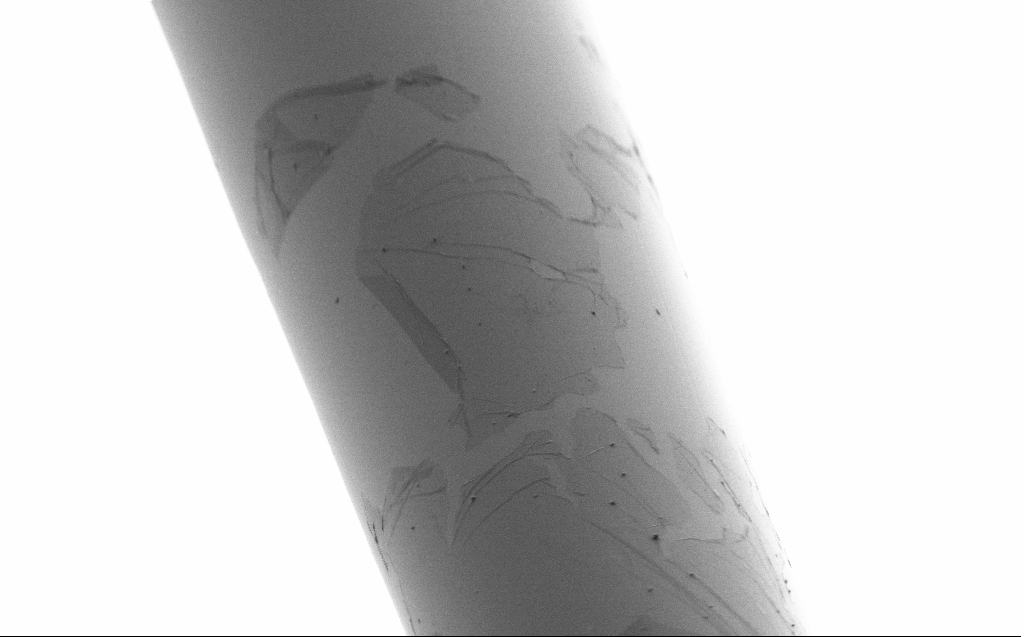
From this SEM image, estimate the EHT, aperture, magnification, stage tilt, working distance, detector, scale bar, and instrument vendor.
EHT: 1 kV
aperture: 30 µm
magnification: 5 K X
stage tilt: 45°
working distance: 4 mm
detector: SE2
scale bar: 10000 nm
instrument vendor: Zeiss